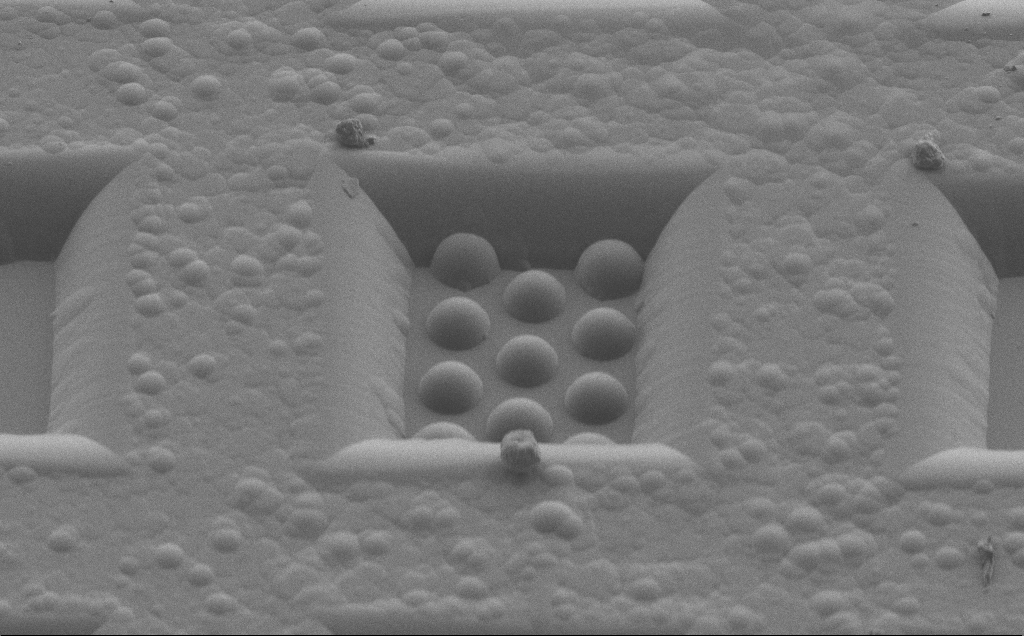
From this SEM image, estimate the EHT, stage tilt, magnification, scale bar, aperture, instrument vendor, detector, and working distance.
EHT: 1.3 kV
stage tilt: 38.2°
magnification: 1.49 K X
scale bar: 20000 nm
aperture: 30 µm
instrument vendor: Zeiss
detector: SE2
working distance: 6 mm